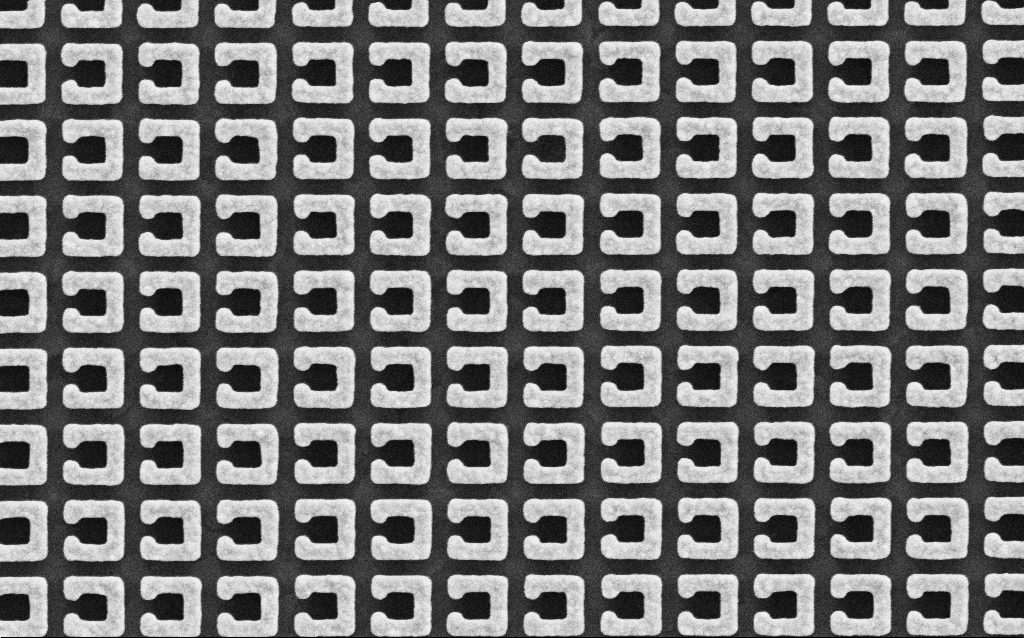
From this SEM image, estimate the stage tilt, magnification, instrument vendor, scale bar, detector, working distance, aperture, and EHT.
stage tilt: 0°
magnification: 61.23 K X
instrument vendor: Zeiss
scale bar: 1000 nm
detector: SE2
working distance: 6.1 mm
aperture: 30 µm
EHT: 5 kV